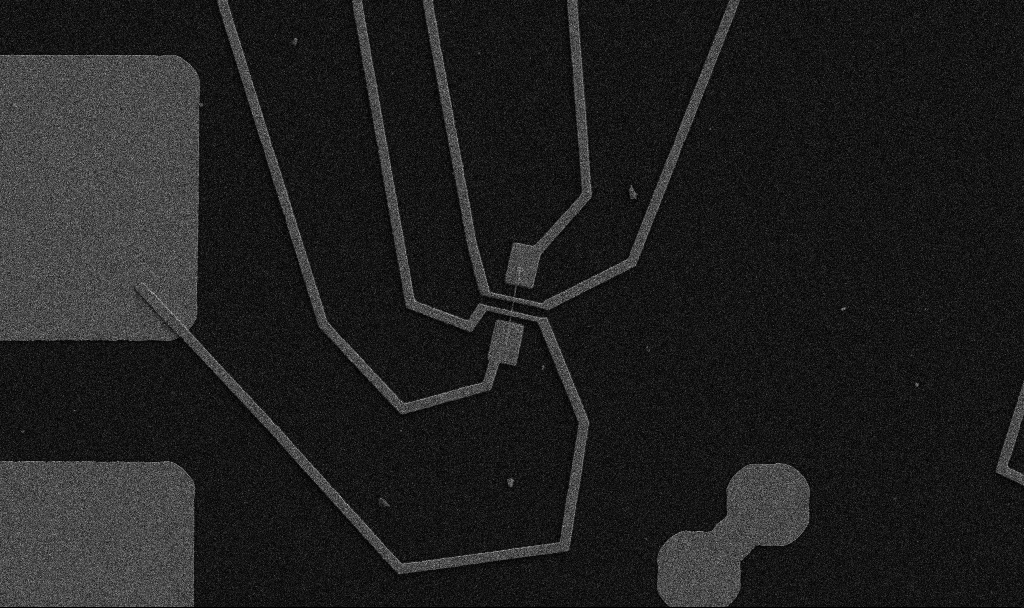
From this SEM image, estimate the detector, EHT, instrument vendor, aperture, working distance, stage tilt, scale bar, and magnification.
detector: SE2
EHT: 5 kV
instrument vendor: Zeiss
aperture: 30 µm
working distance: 10.7 mm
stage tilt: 0°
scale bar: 10000 nm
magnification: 5 K X